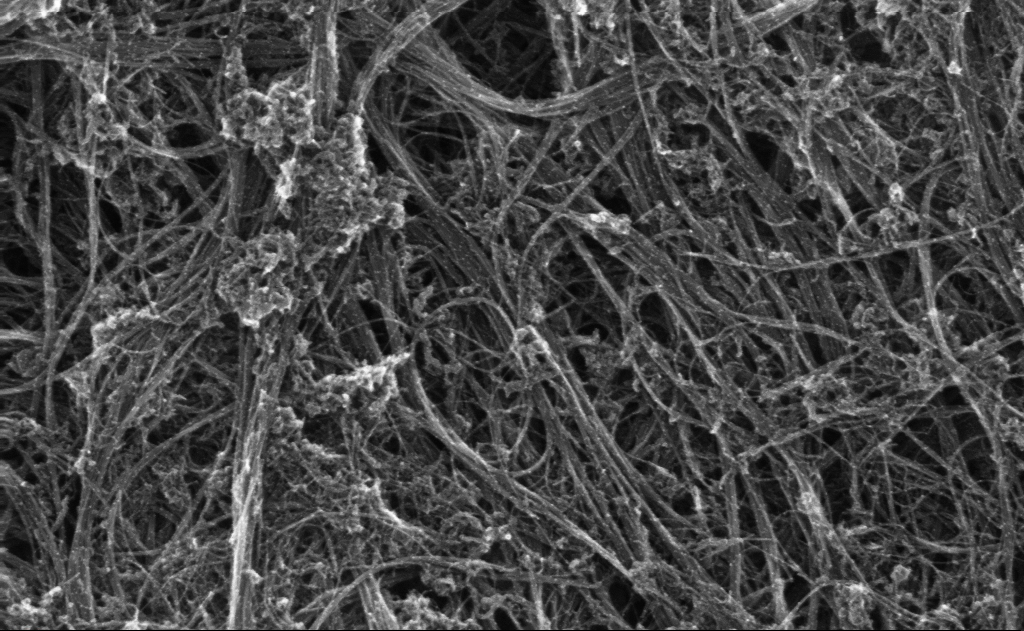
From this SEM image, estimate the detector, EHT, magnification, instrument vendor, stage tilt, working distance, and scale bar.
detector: InLens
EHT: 10 kV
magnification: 173.01 K X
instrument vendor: Zeiss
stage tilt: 0°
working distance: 3 mm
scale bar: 100 nm